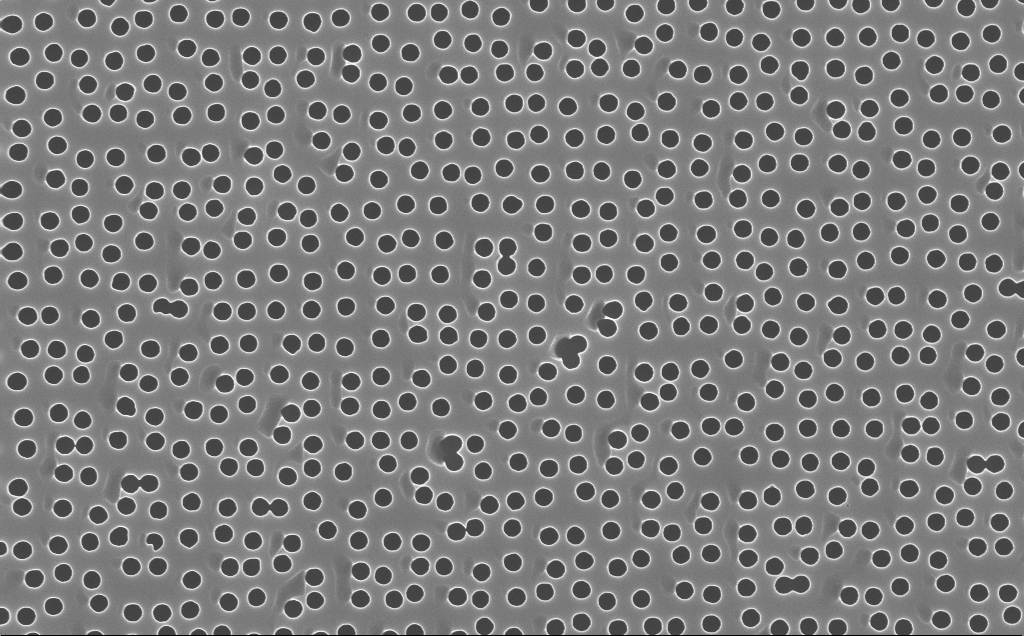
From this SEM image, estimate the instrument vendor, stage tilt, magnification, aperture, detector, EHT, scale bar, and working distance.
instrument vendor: Zeiss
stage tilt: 0°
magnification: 30 K X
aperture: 30 µm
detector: InLens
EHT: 10 kV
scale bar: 1000 nm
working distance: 4 mm